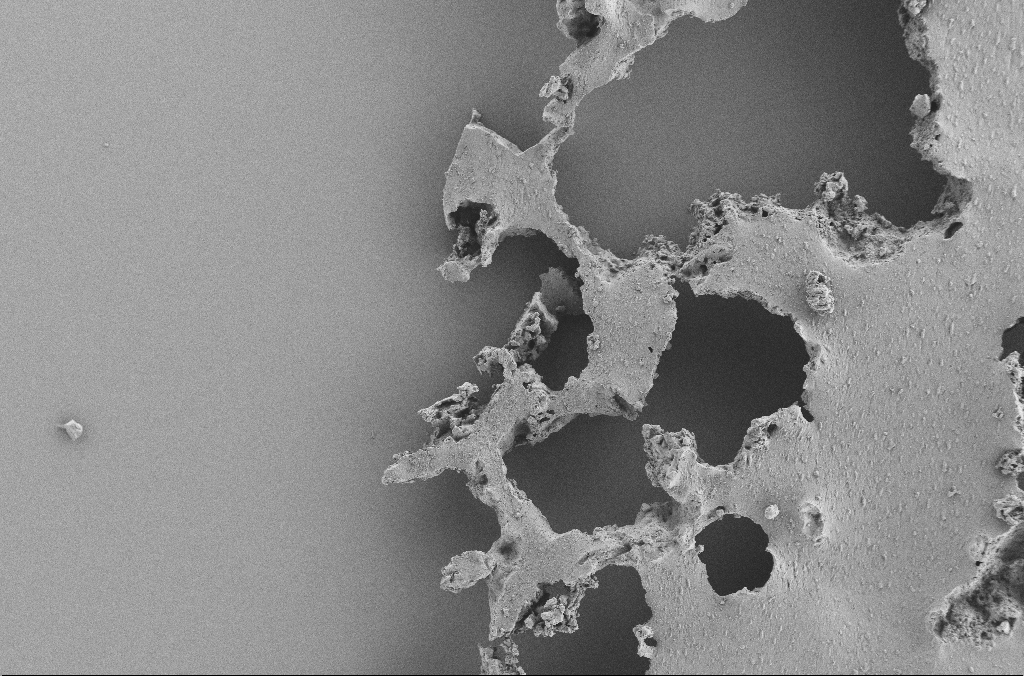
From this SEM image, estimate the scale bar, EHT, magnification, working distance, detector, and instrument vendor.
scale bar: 100000 nm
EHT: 2 kV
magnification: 0.25 K X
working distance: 3.6 mm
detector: SE2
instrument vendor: Zeiss